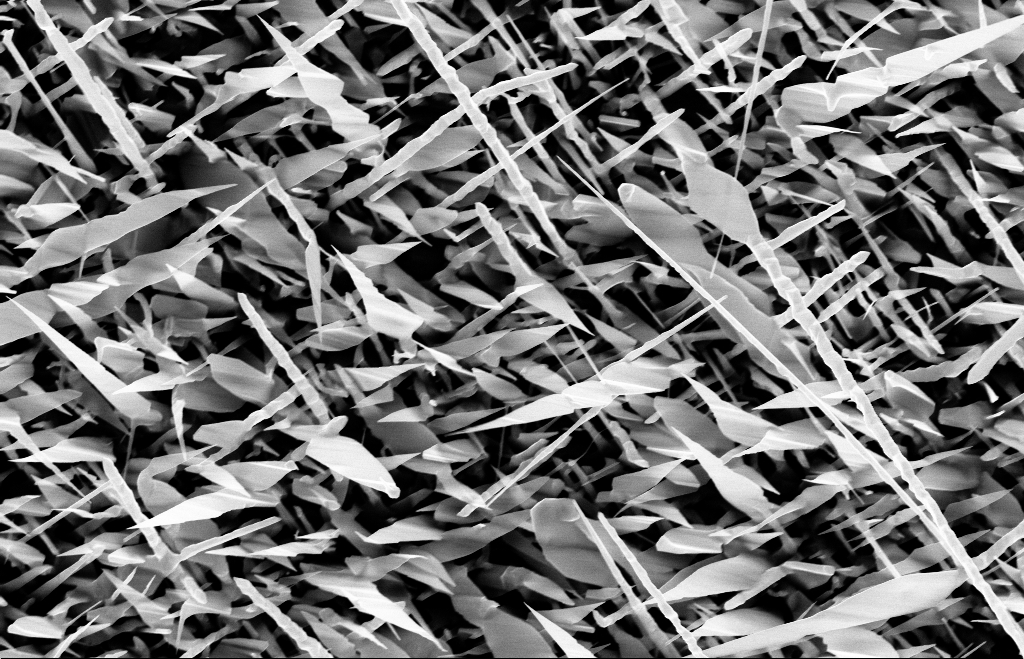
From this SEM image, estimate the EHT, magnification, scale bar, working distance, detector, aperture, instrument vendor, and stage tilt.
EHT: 10 kV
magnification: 20 K X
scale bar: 1000 nm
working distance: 8 mm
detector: InLens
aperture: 30 µm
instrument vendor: Zeiss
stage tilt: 0°